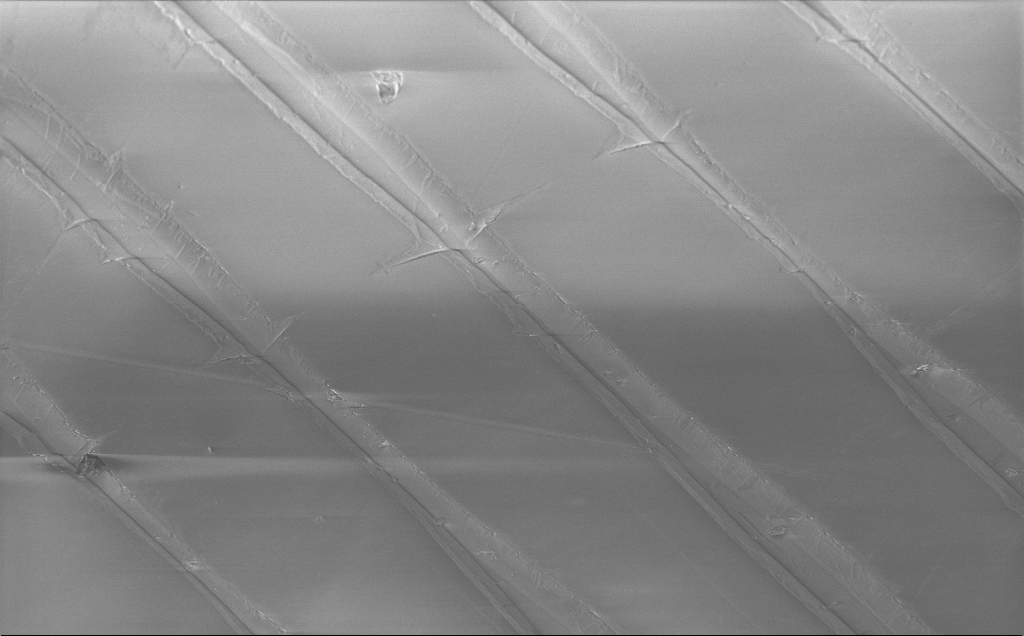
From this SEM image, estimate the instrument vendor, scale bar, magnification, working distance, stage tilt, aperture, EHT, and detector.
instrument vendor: Zeiss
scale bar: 20000 nm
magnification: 0.844 K X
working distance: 9 mm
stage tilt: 45°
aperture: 30 µm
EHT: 3 kV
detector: InLens